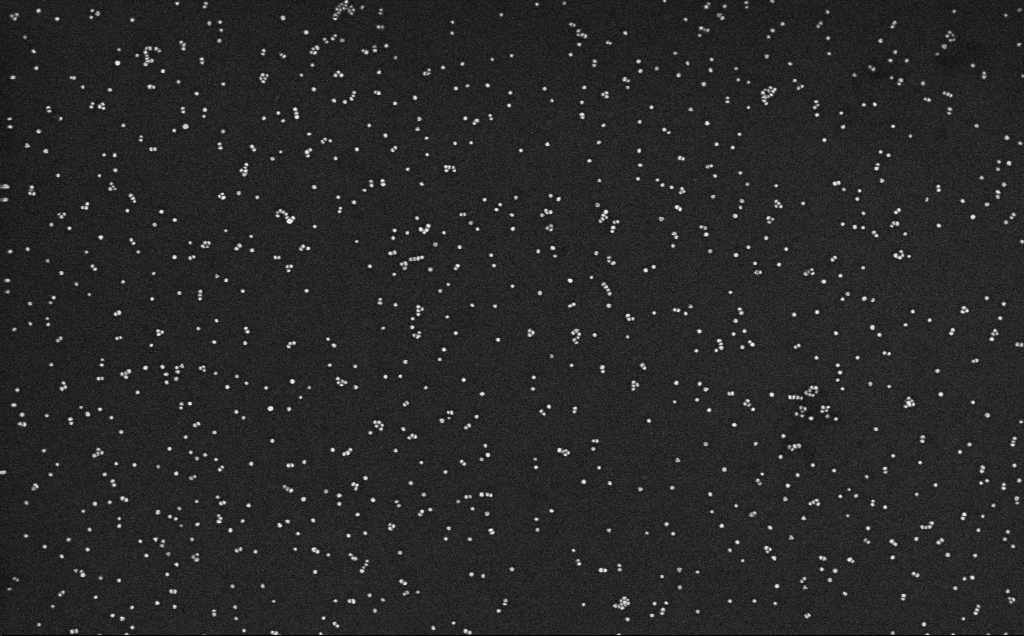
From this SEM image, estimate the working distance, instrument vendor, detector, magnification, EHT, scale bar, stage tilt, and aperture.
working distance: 3.3 mm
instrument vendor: Zeiss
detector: InLens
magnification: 100 K X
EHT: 10 kV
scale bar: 200 nm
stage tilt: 0°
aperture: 30 µm